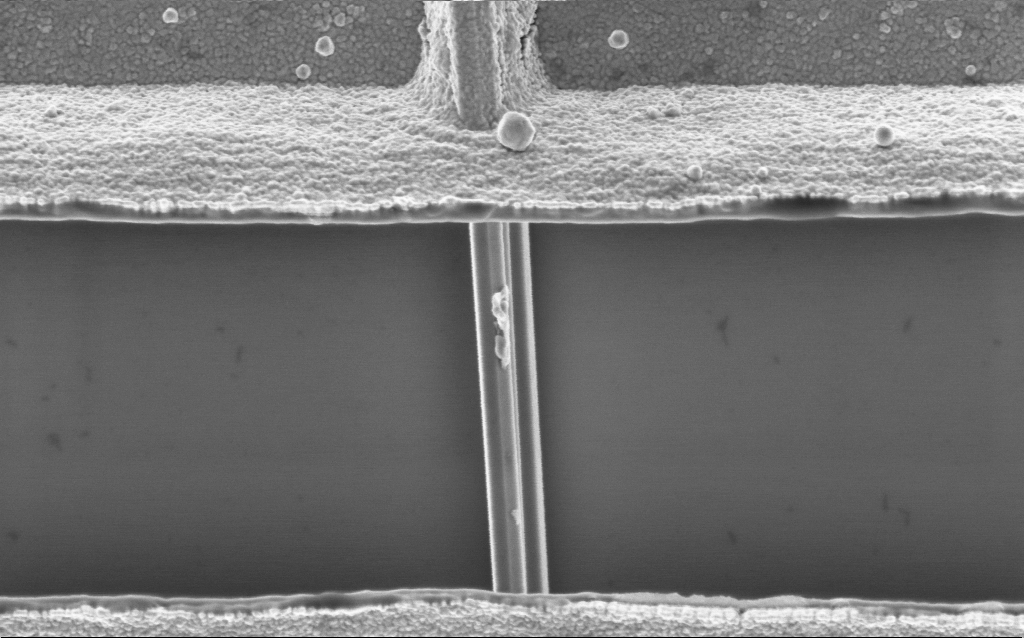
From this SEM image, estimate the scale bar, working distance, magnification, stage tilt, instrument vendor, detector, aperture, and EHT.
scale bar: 1000 nm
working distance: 7.7 mm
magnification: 63.69 K X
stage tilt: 0°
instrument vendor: Zeiss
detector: InLens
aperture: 30 µm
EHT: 2 kV